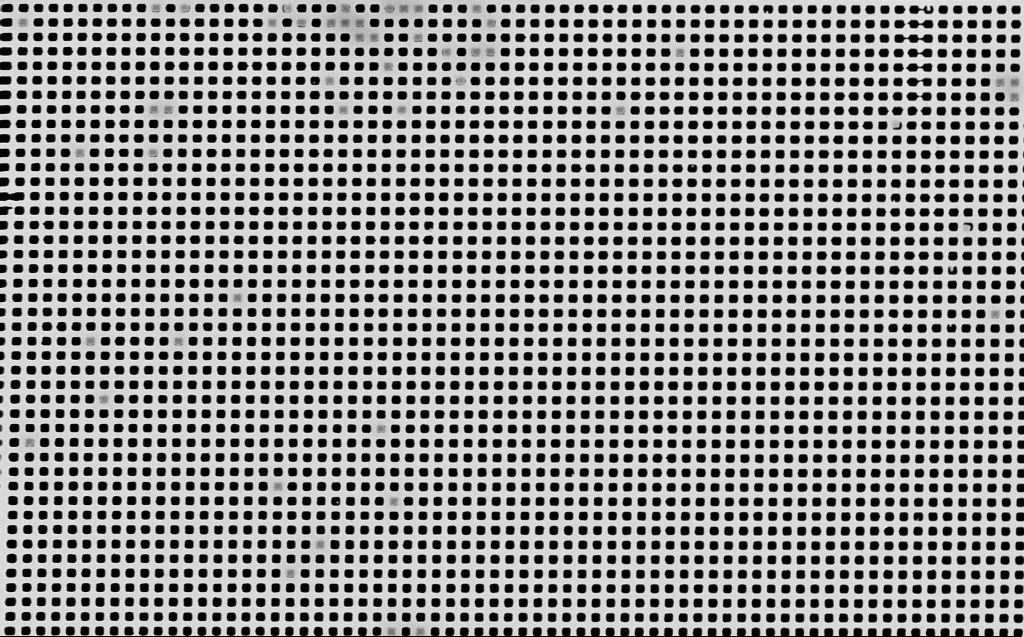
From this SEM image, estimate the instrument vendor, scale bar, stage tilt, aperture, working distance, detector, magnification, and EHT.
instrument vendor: Zeiss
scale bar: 2000 nm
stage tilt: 0°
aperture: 30 µm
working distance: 7 mm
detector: InLens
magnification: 10.81 K X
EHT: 10 kV